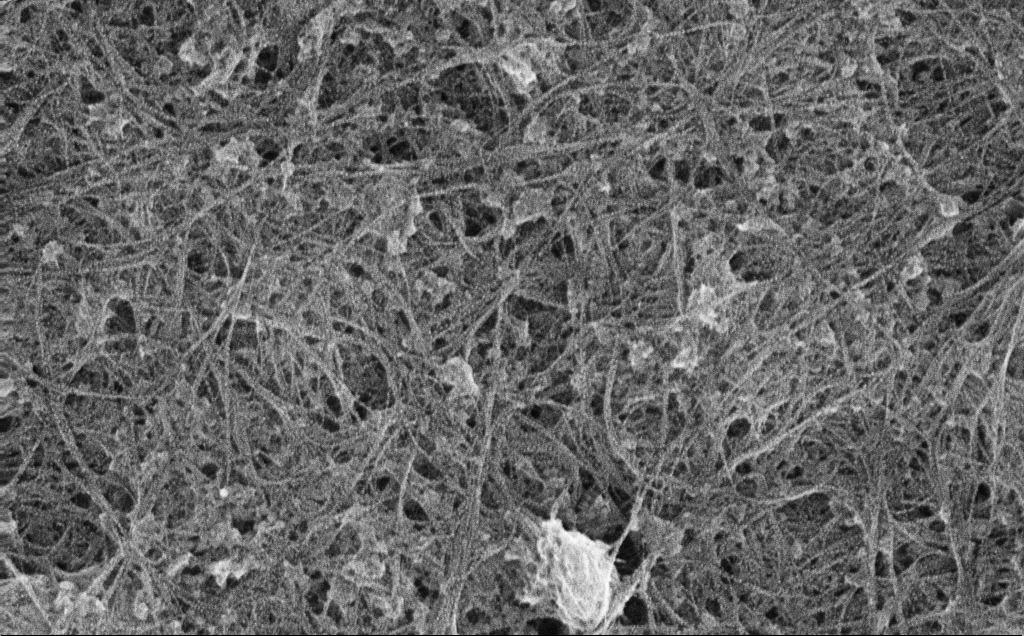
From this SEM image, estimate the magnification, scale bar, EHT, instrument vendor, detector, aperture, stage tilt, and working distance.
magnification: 40.95 K X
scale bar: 1000 nm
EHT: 10 kV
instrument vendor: Zeiss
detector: InLens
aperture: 30 µm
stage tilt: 0°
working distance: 3 mm